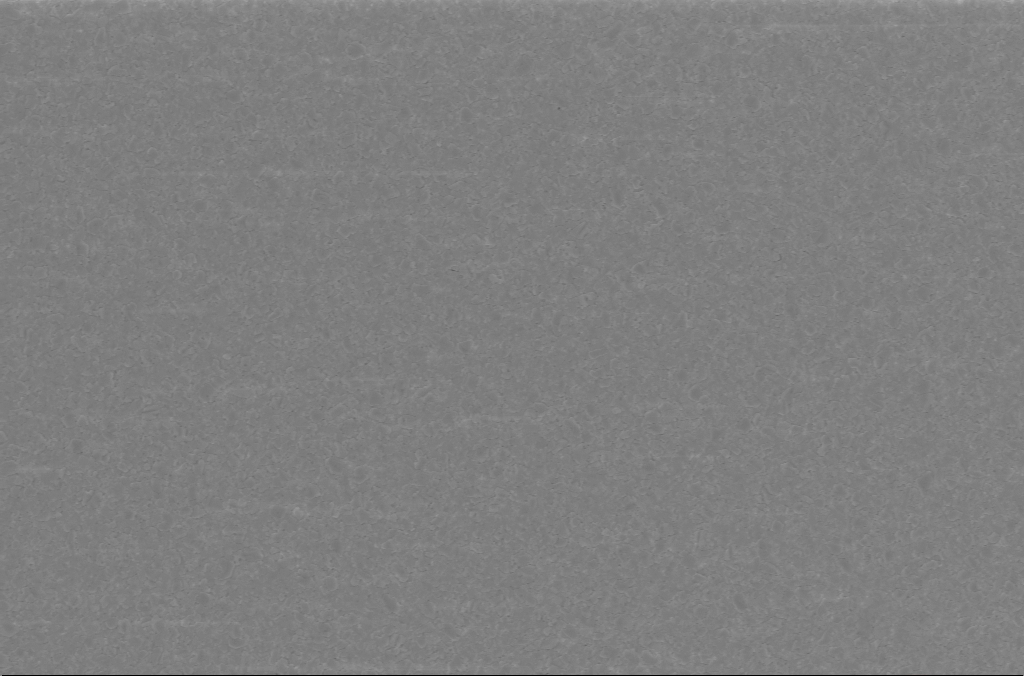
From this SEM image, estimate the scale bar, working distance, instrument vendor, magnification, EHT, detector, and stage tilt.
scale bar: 2000 nm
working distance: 3 mm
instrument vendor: Zeiss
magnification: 10 K X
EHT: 5 kV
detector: InLens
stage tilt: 0°